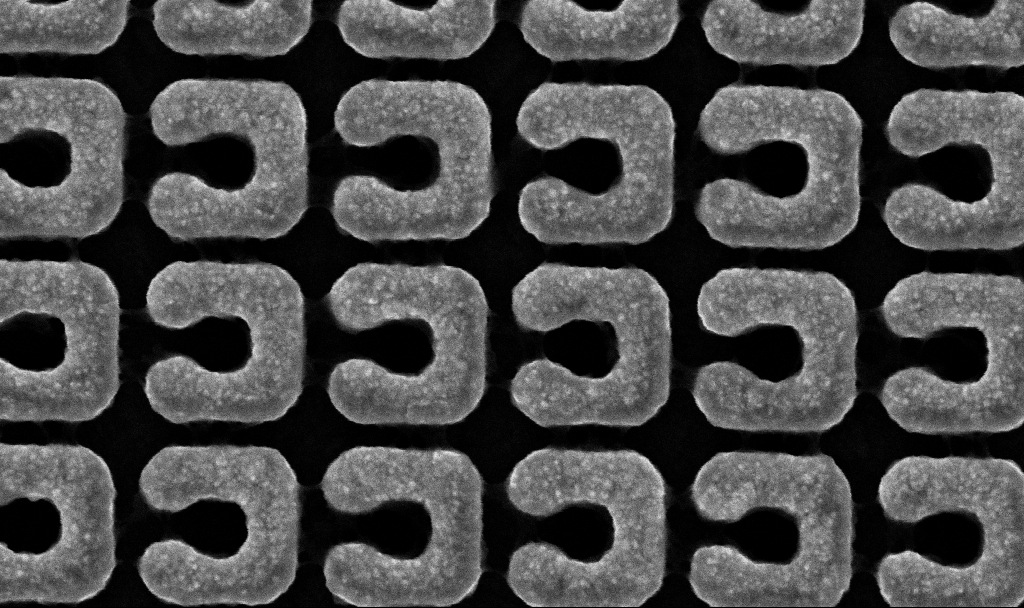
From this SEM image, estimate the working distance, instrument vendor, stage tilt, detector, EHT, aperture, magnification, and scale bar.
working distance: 4.2 mm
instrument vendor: Zeiss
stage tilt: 0°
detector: InLens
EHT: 5 kV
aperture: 30 µm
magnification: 147.65 K X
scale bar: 200 nm